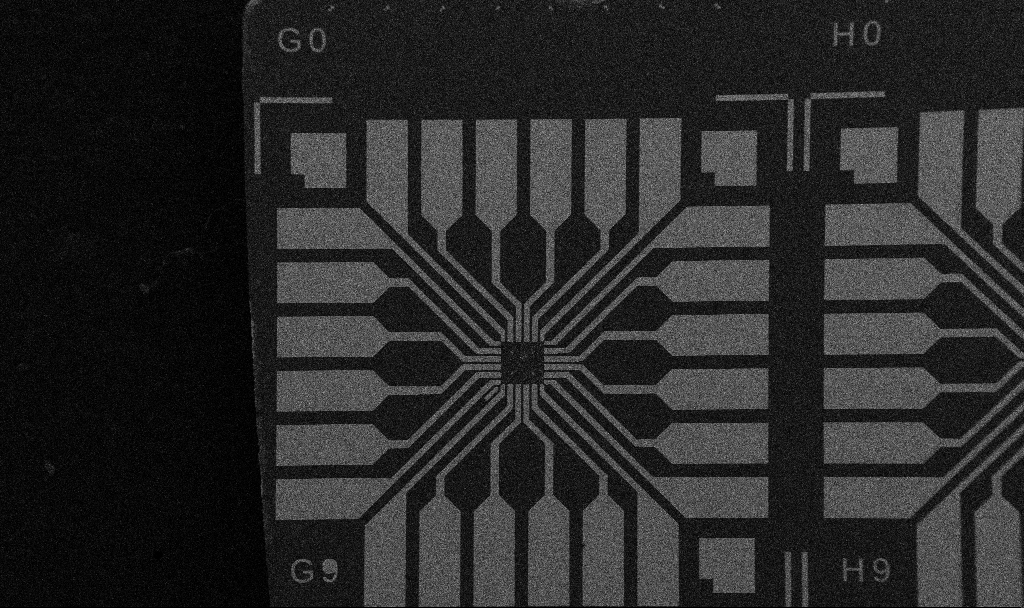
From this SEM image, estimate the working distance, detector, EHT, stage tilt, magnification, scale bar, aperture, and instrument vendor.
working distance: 10.7 mm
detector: SE2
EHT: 5 kV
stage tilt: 0°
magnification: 0.1 K X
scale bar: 200000 nm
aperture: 30 µm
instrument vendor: Zeiss